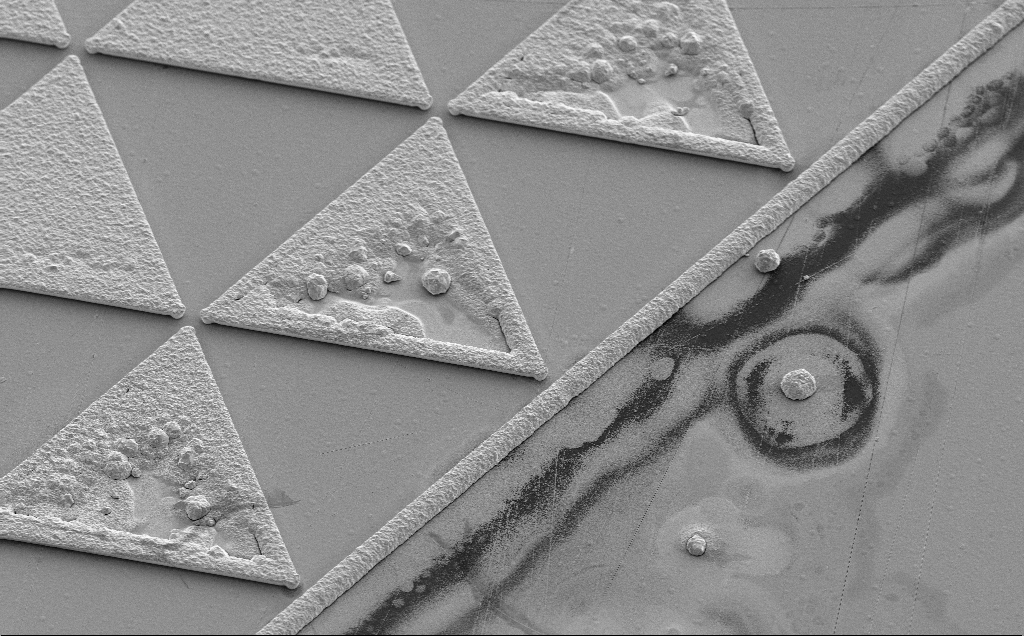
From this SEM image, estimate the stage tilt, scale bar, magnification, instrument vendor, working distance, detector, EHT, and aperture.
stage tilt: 35°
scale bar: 20000 nm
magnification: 2.26 K X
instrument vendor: Zeiss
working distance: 13 mm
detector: SE2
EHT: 5 kV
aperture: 30 µm